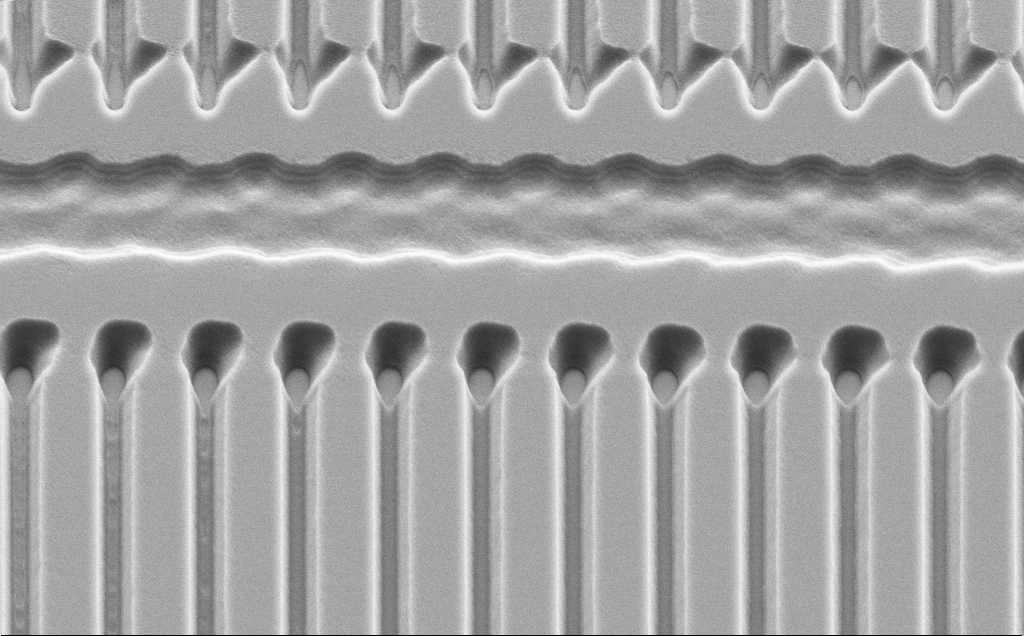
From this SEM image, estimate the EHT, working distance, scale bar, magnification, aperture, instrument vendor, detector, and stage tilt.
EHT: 5 kV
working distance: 10 mm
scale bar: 2000 nm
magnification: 8.39 K X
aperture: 30 µm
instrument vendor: Zeiss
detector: SE2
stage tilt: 45°